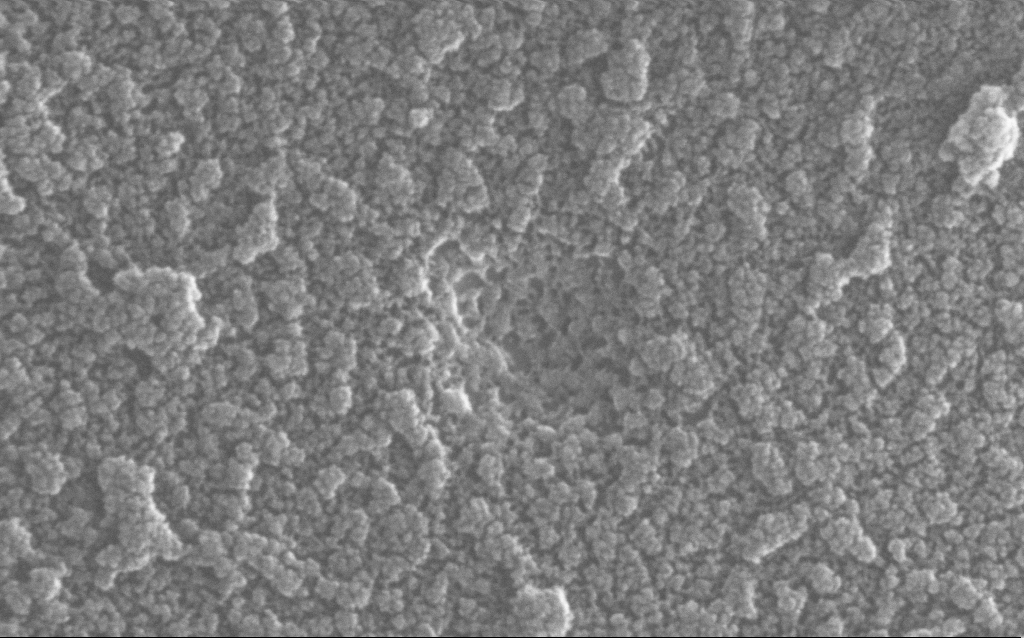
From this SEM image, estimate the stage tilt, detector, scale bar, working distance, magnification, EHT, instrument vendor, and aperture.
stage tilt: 0°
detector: InLens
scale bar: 100 nm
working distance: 1.6 mm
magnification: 500 K X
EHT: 20 kV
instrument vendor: Zeiss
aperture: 30 µm